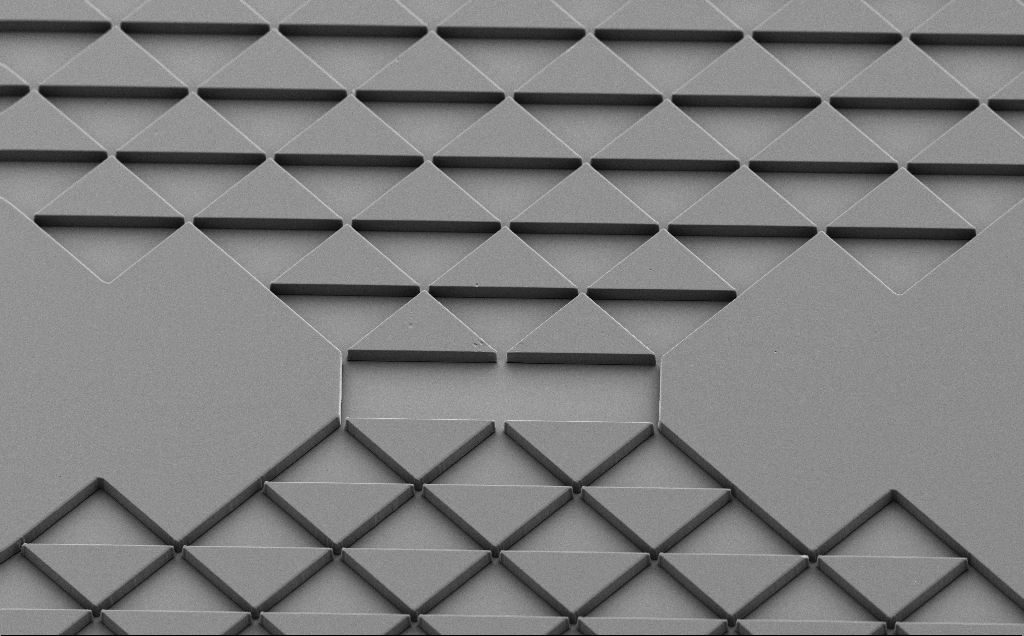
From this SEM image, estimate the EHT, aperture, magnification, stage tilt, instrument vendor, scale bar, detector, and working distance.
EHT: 5 kV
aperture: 30 µm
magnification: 0.619 K X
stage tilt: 40°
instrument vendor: Zeiss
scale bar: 100000 nm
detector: SE2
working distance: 9 mm